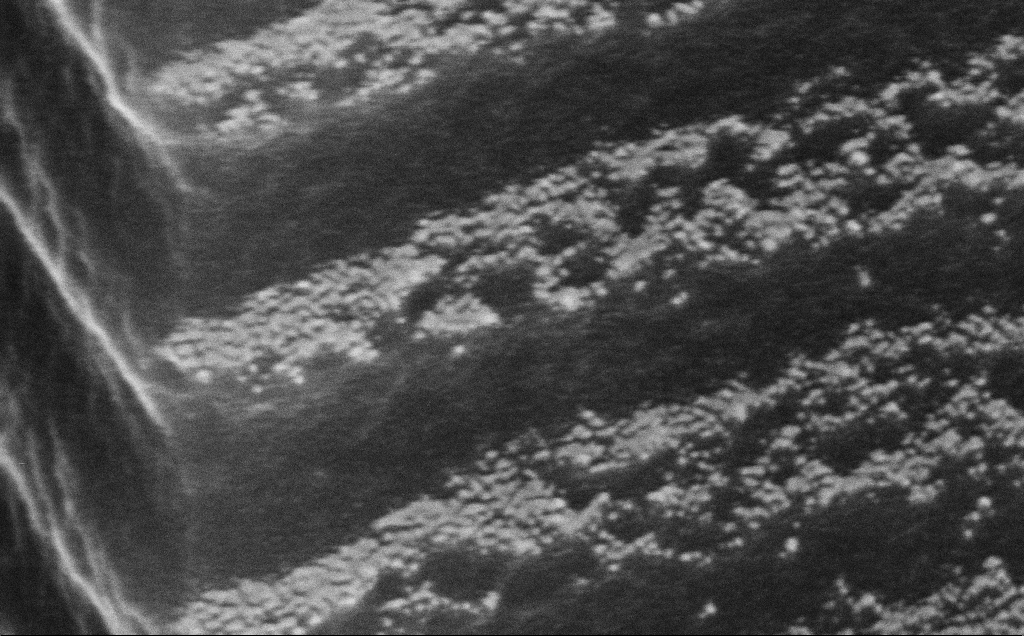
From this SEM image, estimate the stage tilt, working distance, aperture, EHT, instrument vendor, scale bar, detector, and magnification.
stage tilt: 60°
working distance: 5 mm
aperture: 30 µm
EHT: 10 kV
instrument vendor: Zeiss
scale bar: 100 nm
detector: InLens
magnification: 269.51 K X